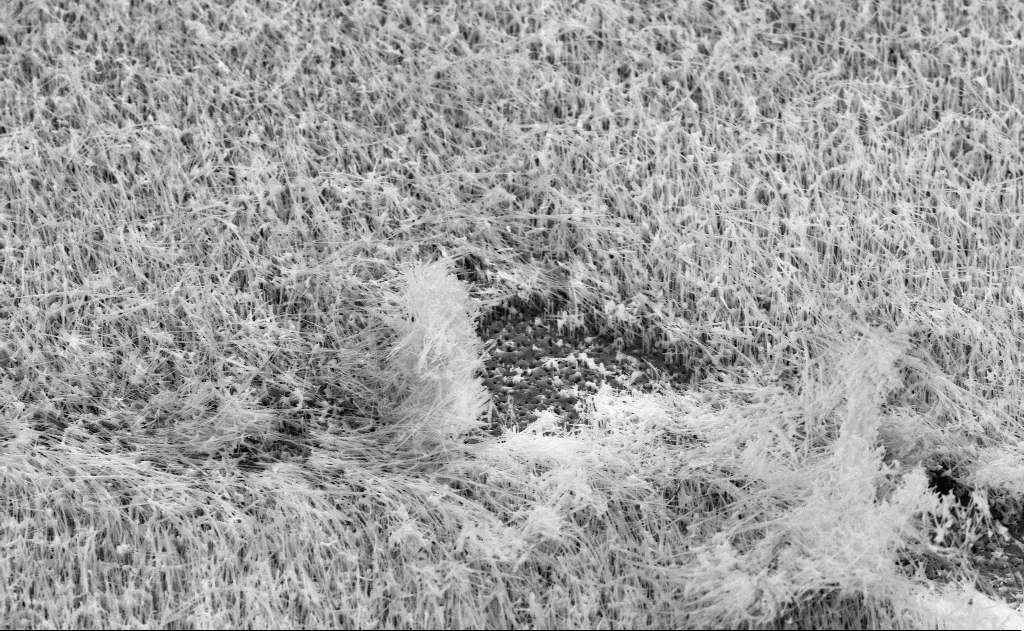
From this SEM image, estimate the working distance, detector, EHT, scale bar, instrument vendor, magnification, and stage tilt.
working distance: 14 mm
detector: SE2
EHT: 10 kV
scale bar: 2000 nm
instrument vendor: Zeiss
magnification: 10 K X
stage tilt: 45°